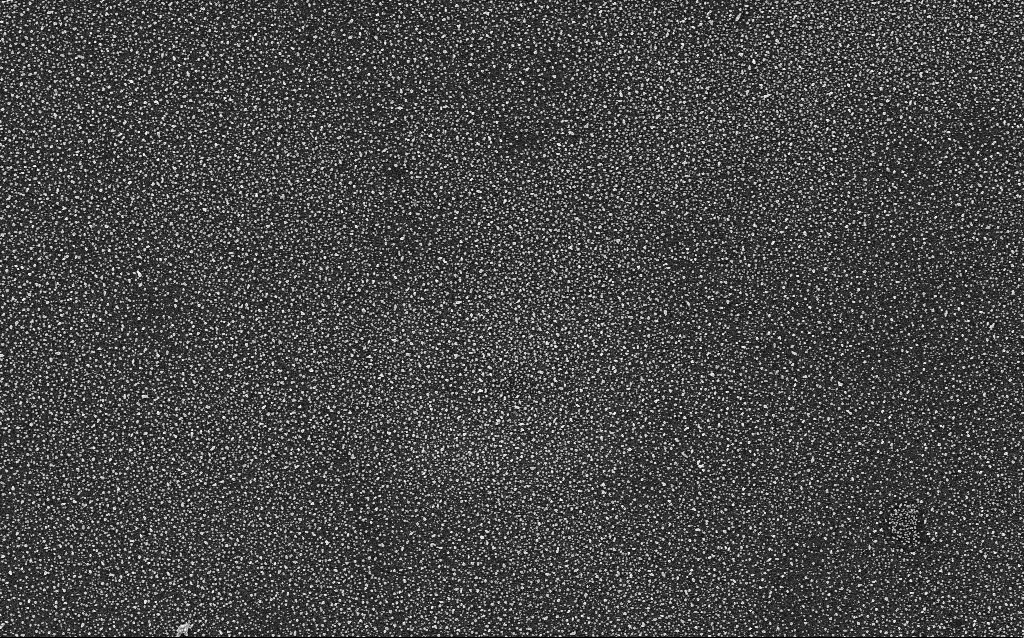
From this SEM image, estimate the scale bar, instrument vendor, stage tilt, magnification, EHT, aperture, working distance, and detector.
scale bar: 2000 nm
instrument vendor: Zeiss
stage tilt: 0°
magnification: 10 K X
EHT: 5 kV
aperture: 30 µm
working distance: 2.1 mm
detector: InLens